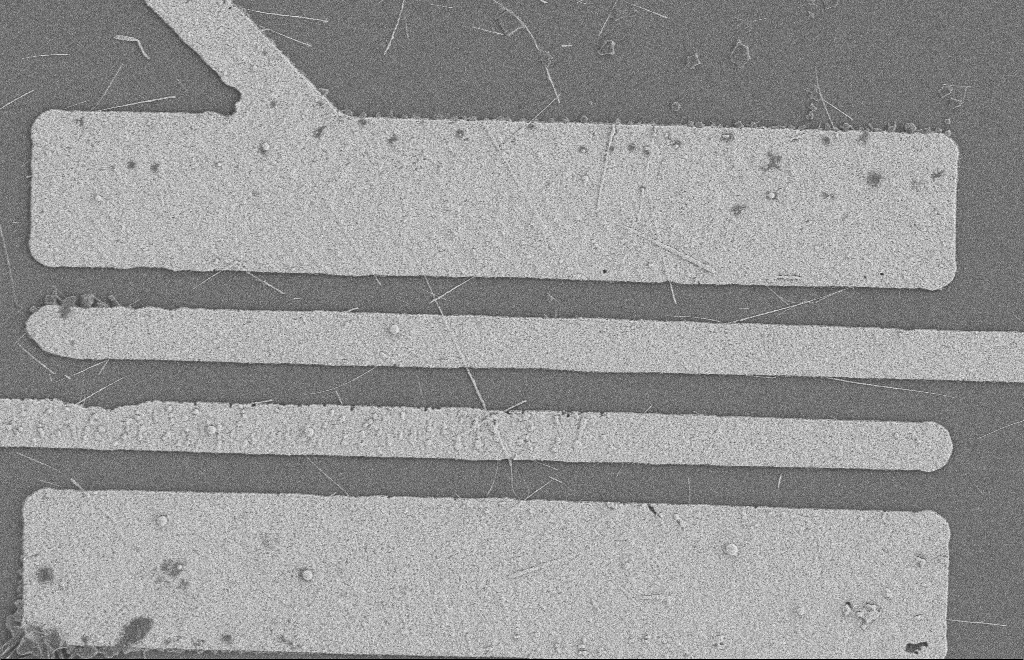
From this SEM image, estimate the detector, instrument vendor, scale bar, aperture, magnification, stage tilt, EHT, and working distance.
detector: SE2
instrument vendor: Zeiss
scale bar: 2000 nm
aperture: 20 µm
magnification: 5.58 K X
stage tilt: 0°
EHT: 2 kV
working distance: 8 mm